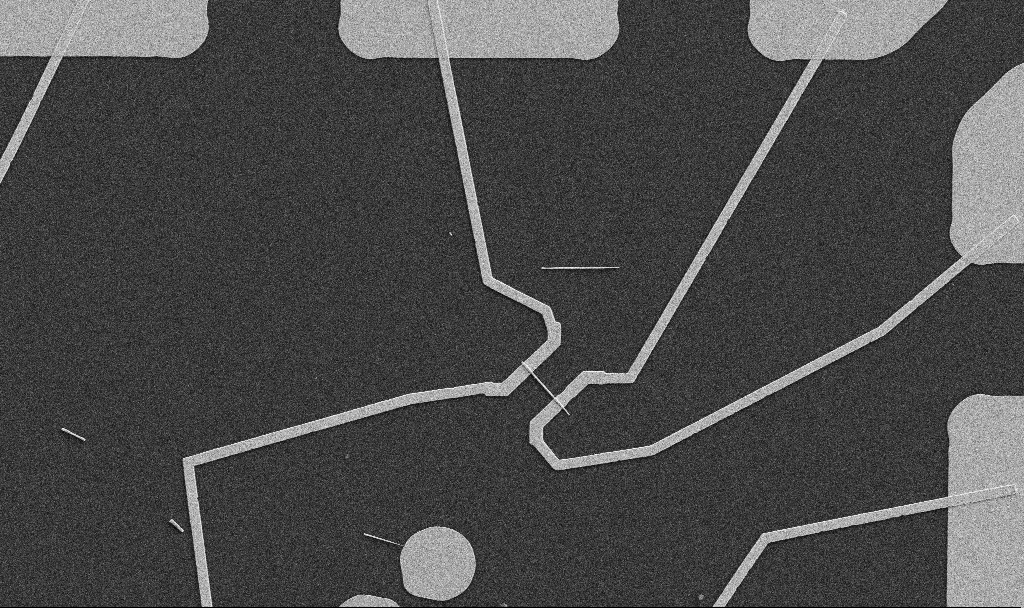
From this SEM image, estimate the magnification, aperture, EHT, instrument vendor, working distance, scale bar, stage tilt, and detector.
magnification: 5 K X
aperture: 30 µm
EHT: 5 kV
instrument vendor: Zeiss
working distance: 10.7 mm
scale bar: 10000 nm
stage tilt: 0°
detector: SE2